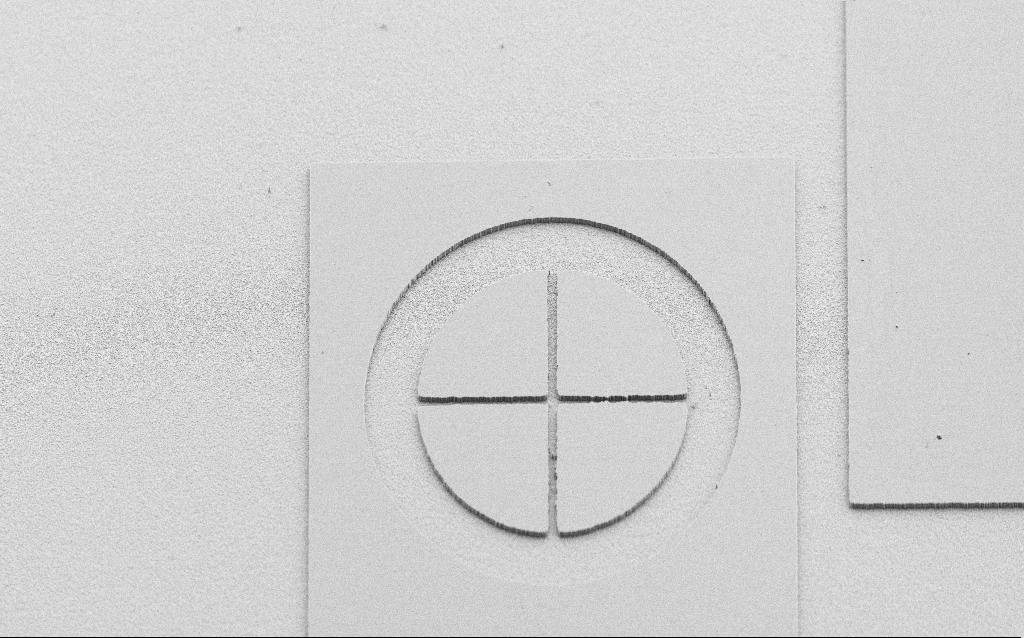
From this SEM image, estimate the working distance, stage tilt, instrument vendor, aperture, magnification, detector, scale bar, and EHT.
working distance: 7 mm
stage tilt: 45°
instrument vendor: Zeiss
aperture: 30 µm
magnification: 0.4 K X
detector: SE2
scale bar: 100000 nm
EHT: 5 kV